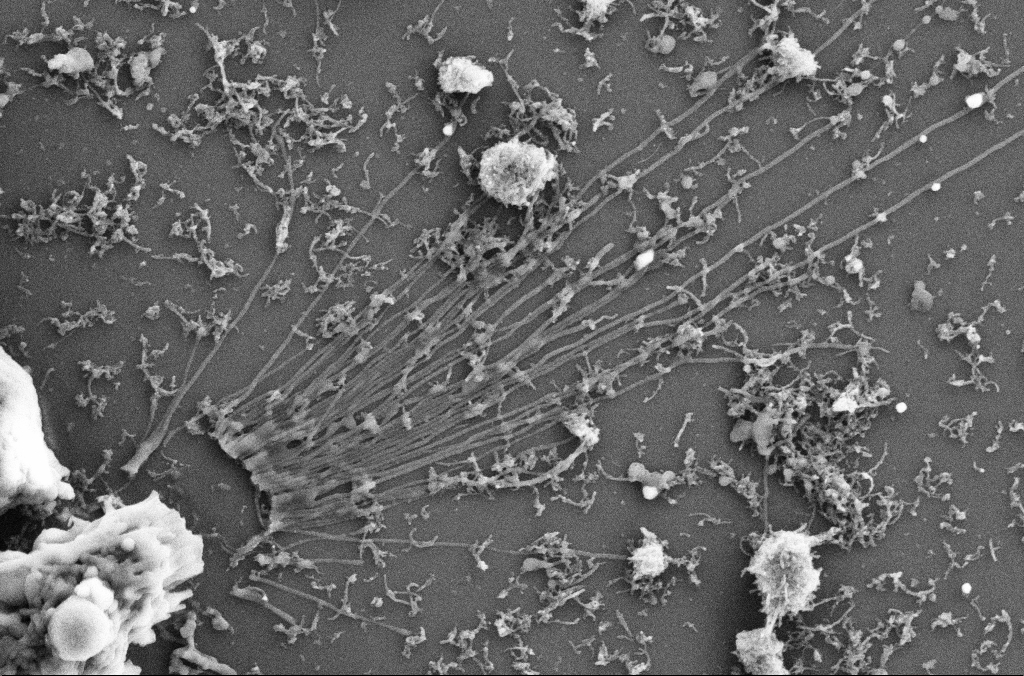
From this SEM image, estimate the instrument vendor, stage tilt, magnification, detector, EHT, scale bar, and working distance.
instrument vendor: Zeiss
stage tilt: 0°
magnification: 20 K X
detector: SE2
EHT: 5 kV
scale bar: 1000 nm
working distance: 4 mm